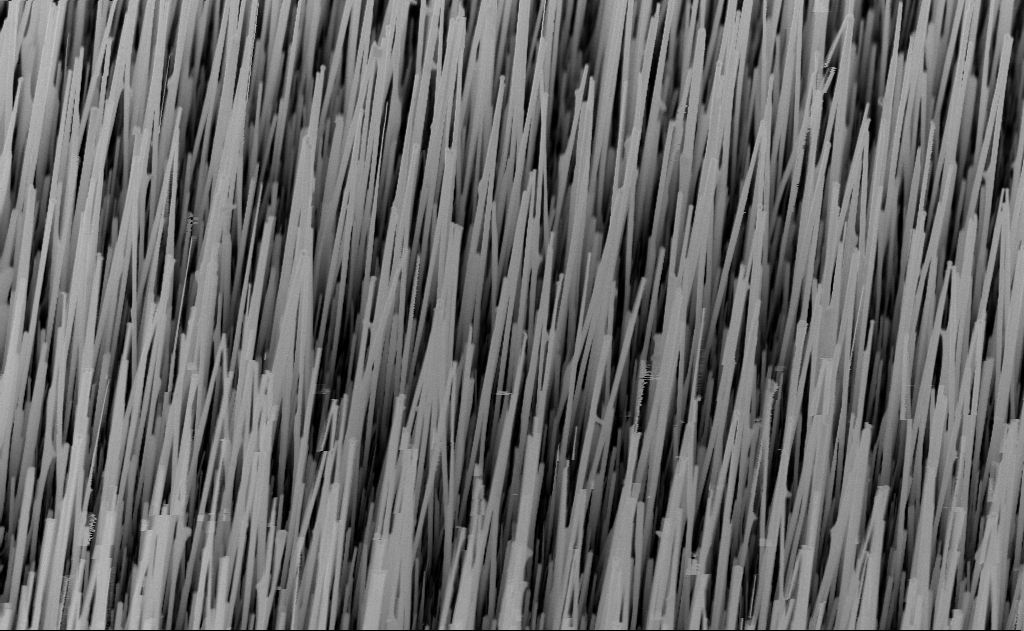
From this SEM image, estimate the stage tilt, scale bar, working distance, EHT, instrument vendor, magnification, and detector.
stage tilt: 30°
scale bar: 1000 nm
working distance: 8 mm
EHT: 10 kV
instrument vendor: Zeiss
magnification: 20 K X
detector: InLens